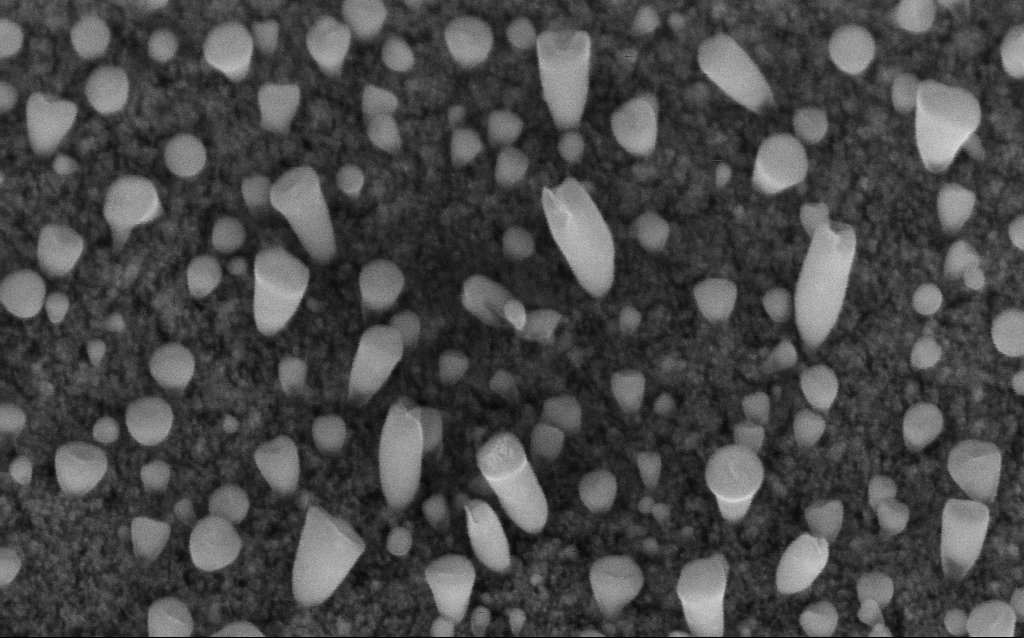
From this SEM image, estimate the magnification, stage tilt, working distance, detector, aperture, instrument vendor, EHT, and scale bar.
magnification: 200 K X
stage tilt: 45°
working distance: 6.2 mm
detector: InLens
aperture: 30 µm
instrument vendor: Zeiss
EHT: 5 kV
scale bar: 100 nm